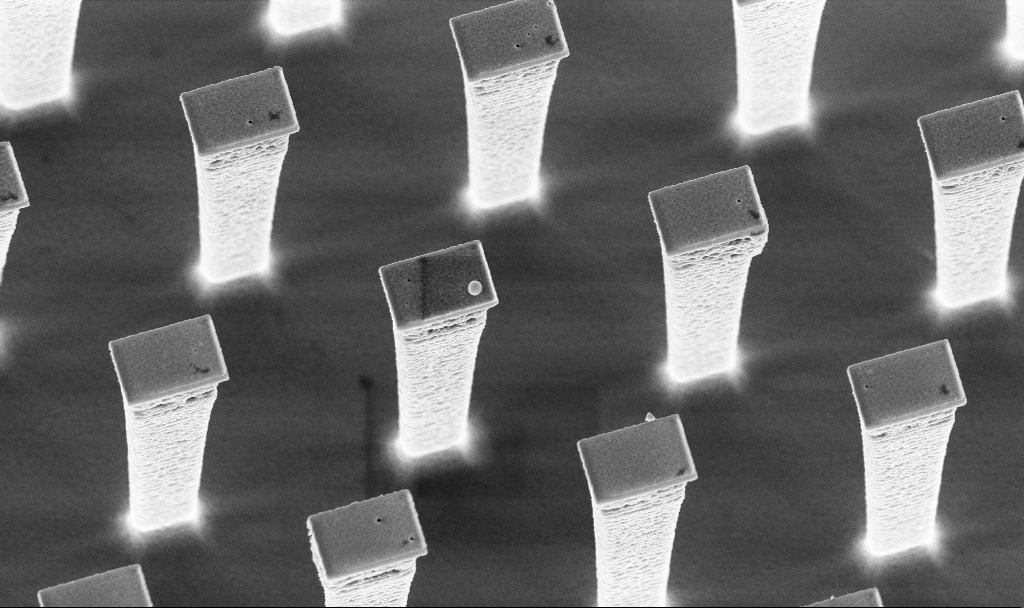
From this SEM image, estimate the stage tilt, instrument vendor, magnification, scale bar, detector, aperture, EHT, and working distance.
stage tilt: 20°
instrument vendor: Zeiss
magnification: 8.63 K X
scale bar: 2000 nm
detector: InLens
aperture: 30 µm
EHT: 5 kV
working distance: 3 mm